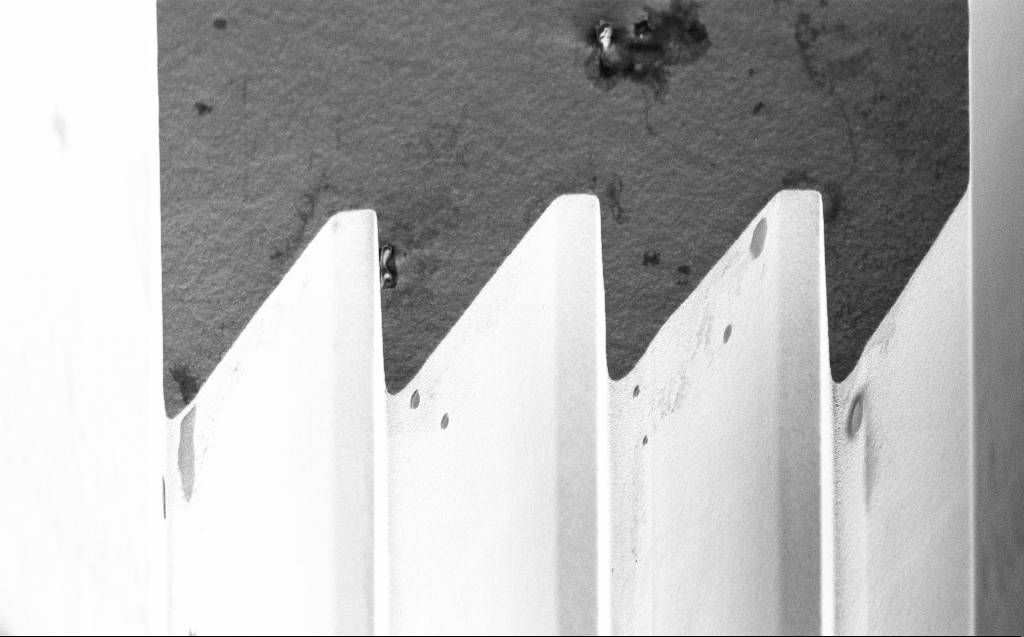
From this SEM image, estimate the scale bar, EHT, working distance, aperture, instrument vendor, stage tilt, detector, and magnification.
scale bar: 2000 nm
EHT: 3 kV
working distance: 5 mm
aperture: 30 µm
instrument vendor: Zeiss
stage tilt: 45°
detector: InLens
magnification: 7.3 K X